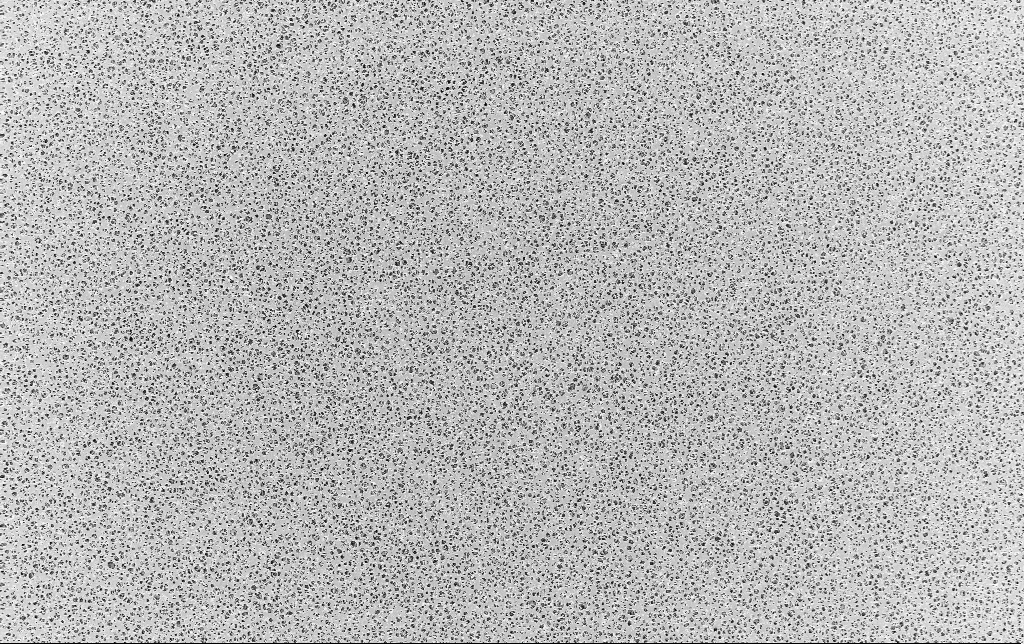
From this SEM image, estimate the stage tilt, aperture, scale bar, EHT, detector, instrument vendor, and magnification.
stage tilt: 0°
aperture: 30 µm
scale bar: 20000 nm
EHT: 2 kV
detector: InLens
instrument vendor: Zeiss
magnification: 1 K X